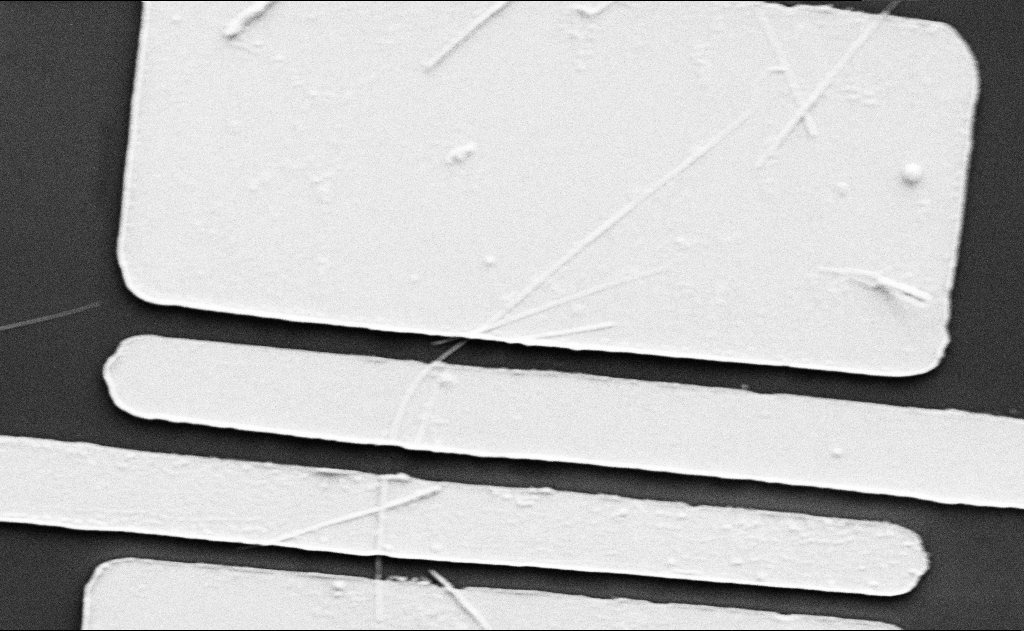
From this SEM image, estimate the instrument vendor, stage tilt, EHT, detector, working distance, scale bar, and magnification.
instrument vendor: Zeiss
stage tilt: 0°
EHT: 5 kV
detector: SE2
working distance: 15 mm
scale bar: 2000 nm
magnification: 10 K X